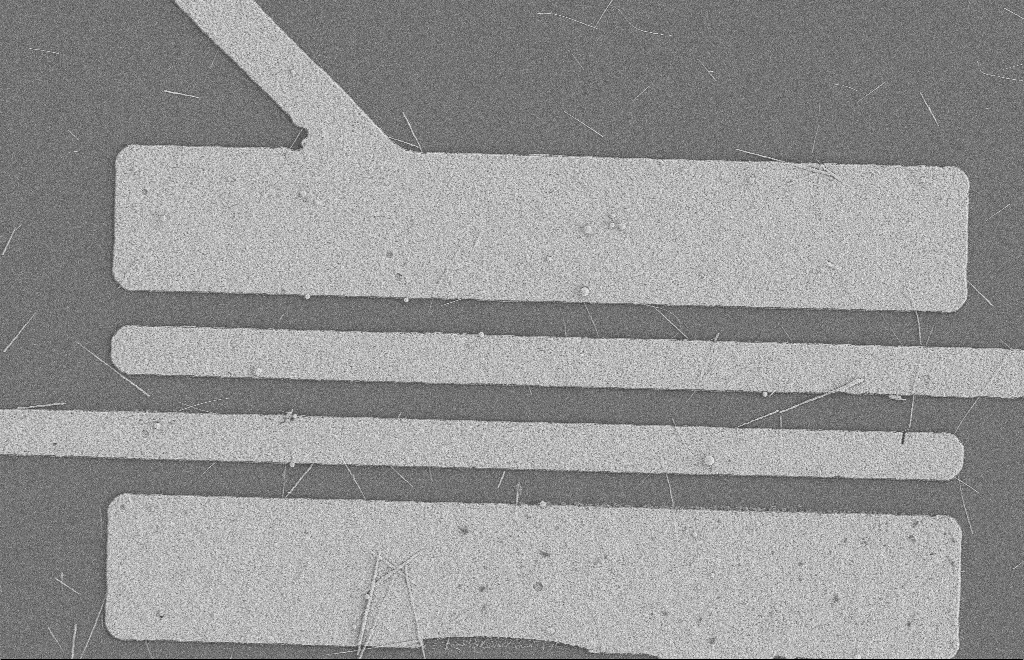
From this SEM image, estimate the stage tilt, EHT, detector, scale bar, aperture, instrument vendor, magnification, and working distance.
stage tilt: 0°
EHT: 2 kV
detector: SE2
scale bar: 2000 nm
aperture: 20 µm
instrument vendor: Zeiss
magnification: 5.15 K X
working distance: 8 mm